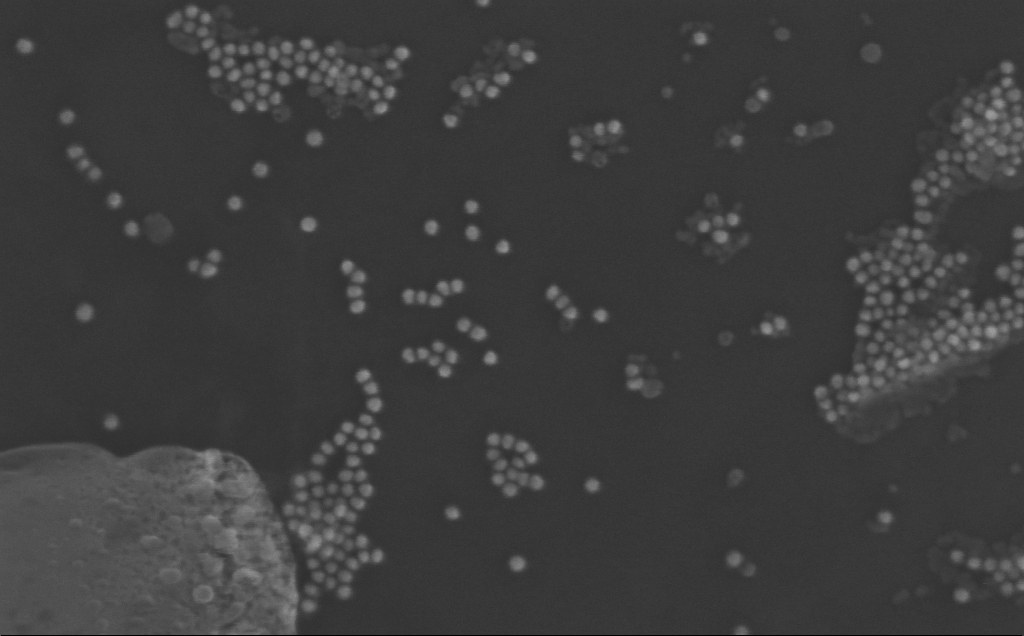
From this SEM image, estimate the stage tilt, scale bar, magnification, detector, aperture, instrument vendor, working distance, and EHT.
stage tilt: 0°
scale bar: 200 nm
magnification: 295.4 K X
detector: InLens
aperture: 30 µm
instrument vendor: Zeiss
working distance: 3 mm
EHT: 10 kV